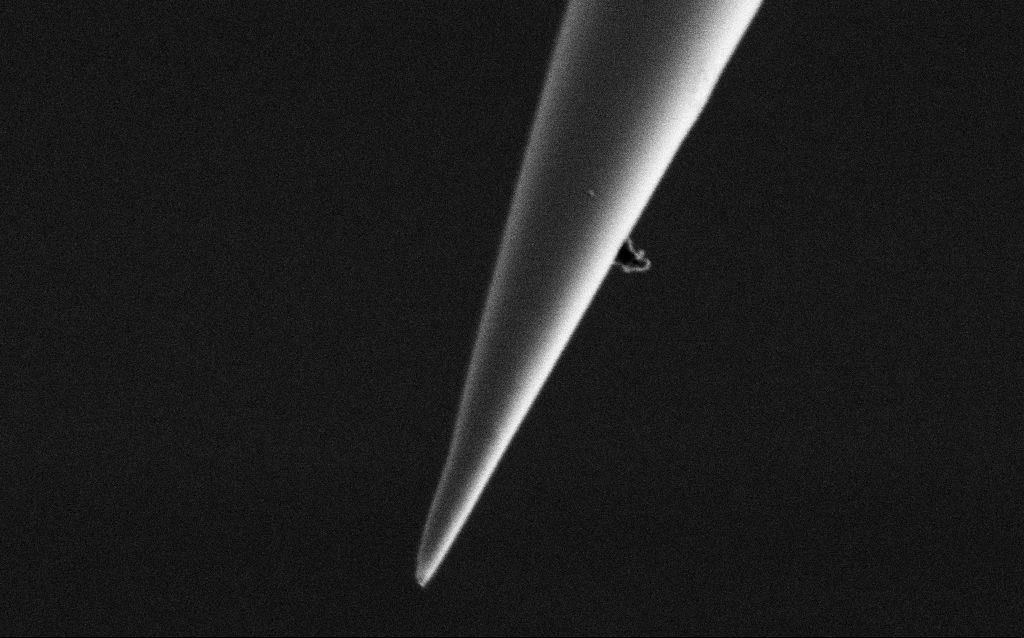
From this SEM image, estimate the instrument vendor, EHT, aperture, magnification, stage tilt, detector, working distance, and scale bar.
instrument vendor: Zeiss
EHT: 1 kV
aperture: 30 µm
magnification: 50 K X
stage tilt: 45°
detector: SE2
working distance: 6.4 mm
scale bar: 1000 nm